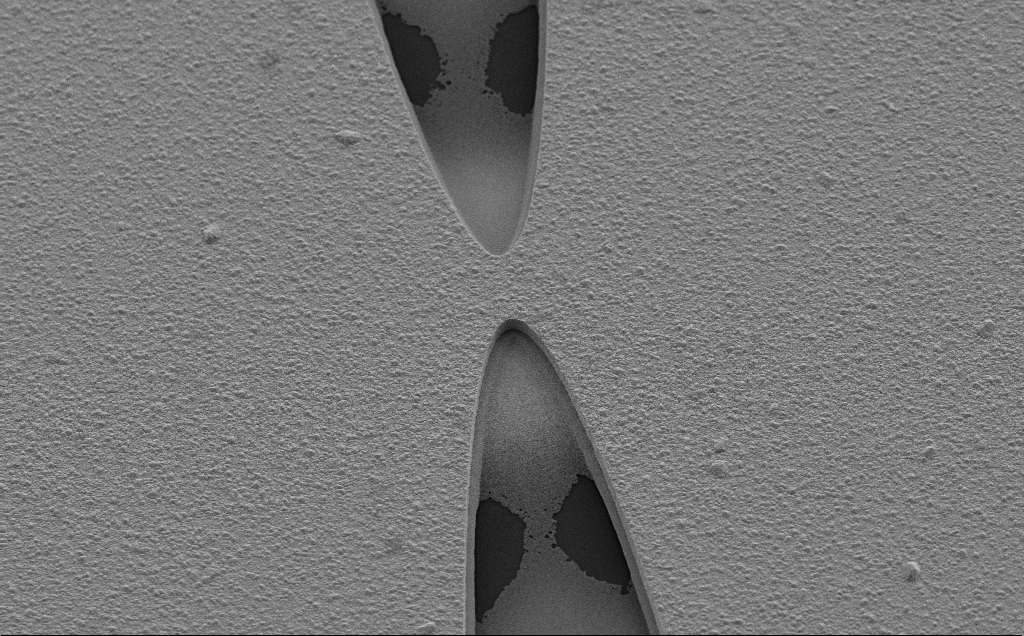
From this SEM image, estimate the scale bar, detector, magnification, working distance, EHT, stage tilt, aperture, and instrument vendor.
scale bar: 10000 nm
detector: SE2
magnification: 2.45 K X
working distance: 8 mm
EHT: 10 kV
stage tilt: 35°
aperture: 30 µm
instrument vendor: Zeiss